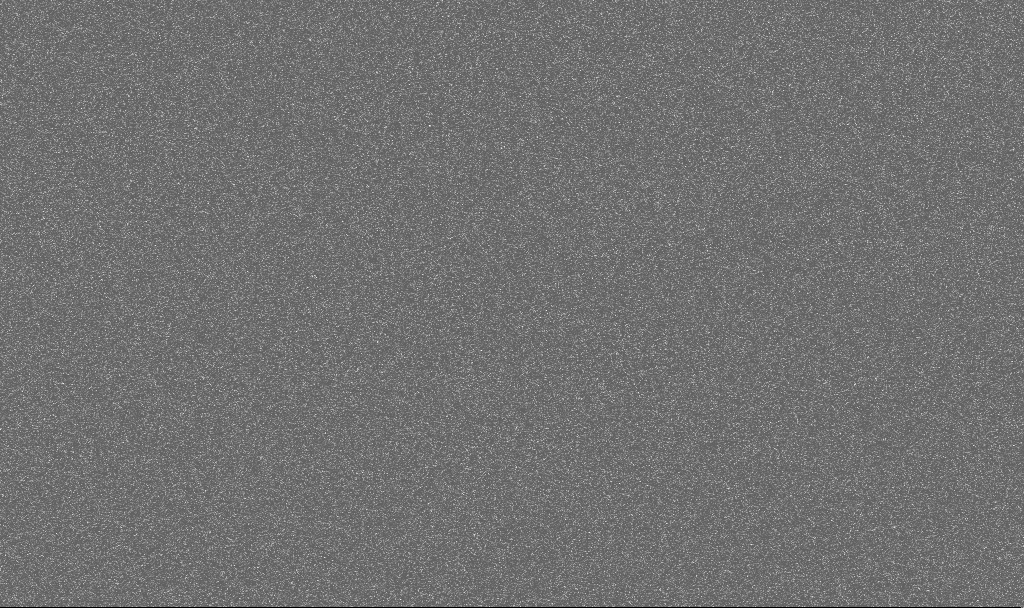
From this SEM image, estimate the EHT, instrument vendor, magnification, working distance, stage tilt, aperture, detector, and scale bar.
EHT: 3 kV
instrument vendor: Zeiss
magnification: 17.4 K X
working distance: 1.3 mm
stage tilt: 0°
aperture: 30 µm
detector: SE2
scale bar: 2000 nm